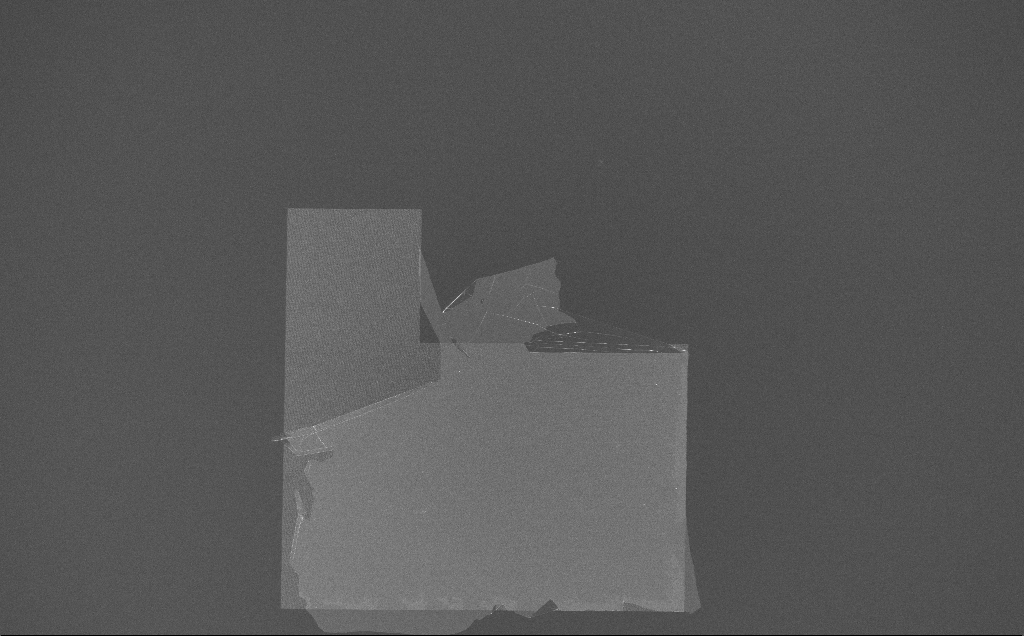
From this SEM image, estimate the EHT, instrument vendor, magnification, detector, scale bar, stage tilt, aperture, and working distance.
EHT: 10 kV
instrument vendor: Zeiss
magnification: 0.2 K X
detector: InLens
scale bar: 100000 nm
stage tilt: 0°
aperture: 30 µm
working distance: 7 mm